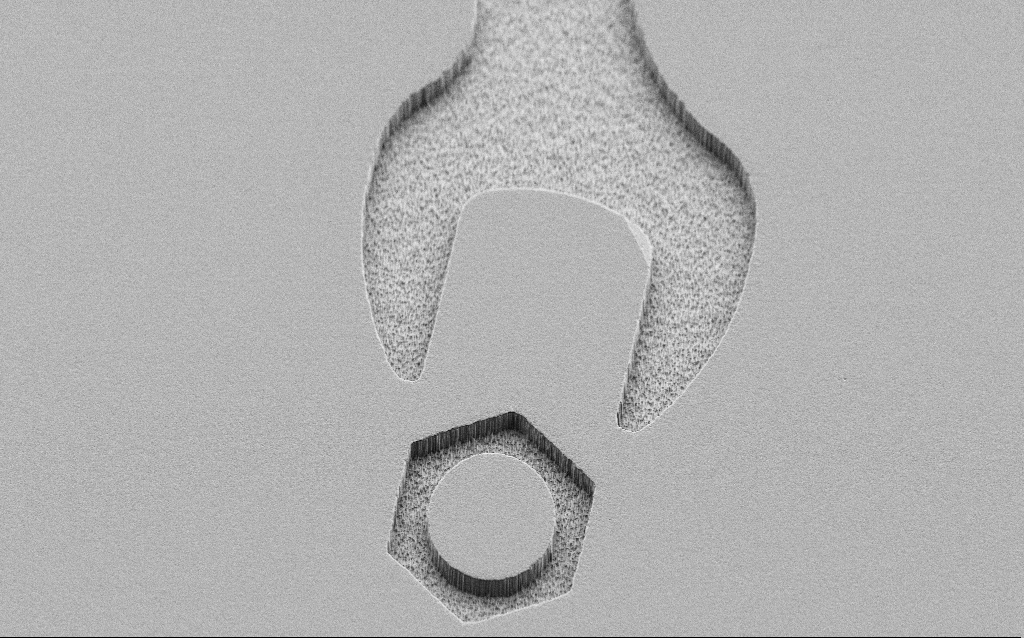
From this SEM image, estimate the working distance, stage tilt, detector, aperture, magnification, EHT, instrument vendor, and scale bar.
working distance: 7 mm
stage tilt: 45°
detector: SE2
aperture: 30 µm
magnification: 1.45 K X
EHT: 3 kV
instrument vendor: Zeiss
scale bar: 10000 nm